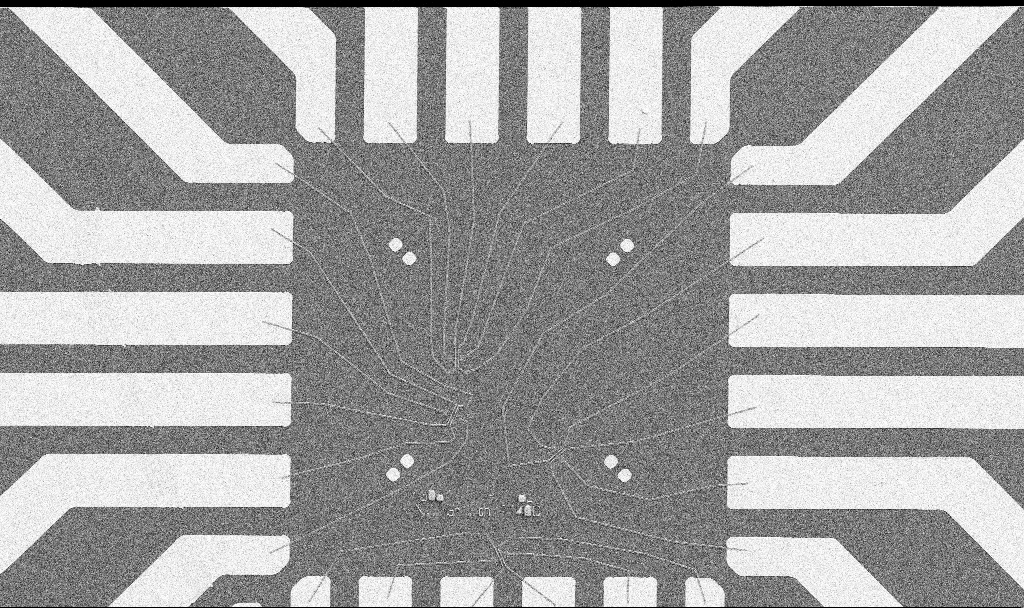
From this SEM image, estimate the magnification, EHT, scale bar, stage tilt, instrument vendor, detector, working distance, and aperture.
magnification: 1 K X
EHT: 5 kV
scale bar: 20000 nm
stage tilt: -0°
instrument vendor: Zeiss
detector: SE2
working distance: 10.7 mm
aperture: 30 µm